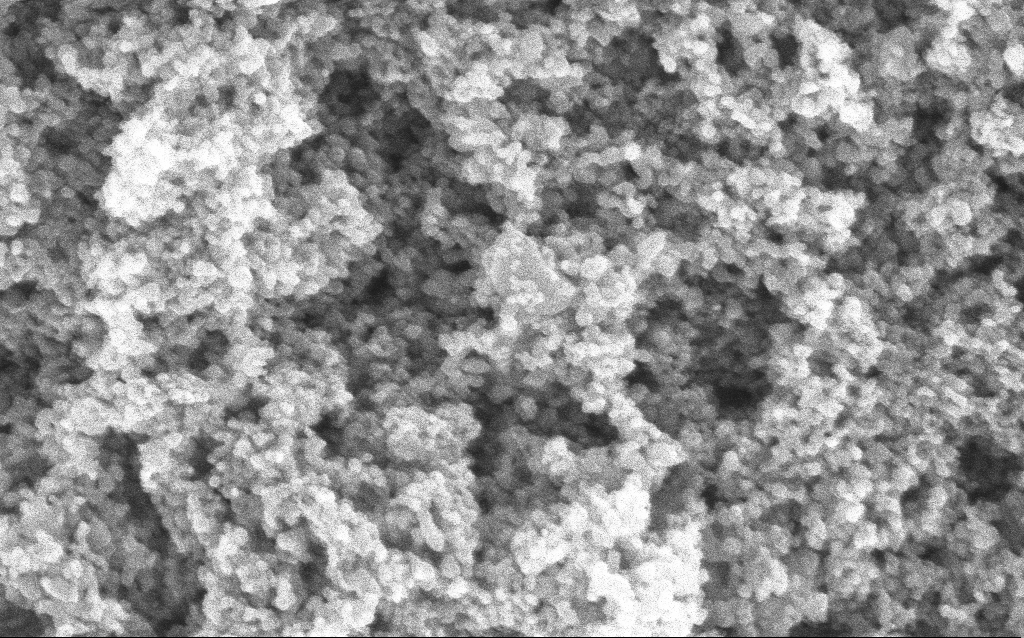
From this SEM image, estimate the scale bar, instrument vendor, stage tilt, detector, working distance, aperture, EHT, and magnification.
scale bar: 100 nm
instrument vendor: Zeiss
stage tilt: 0°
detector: InLens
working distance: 4.4 mm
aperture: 30 µm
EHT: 5 kV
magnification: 162.39 K X